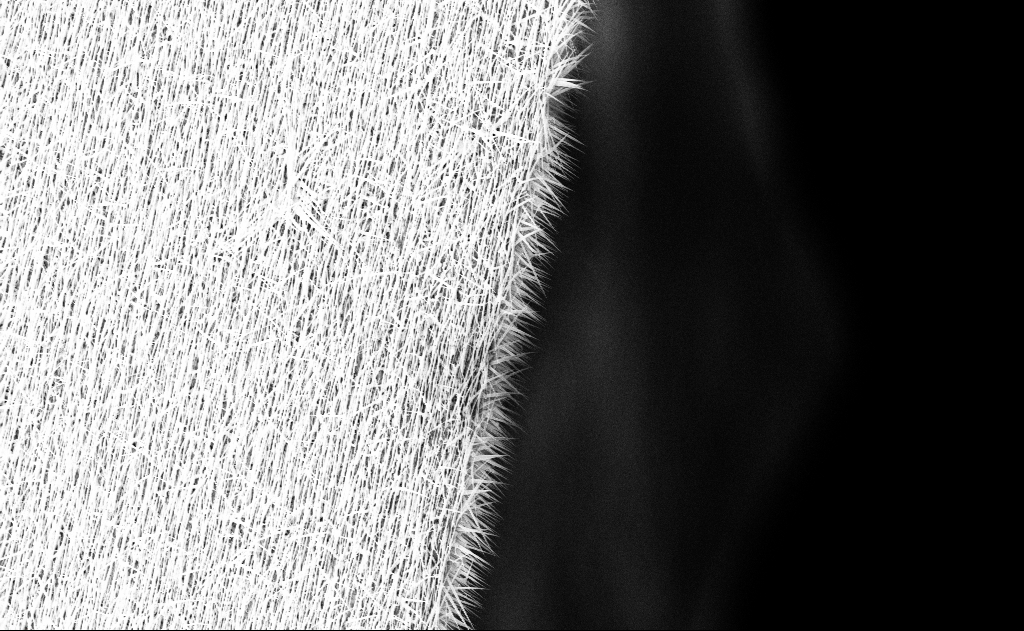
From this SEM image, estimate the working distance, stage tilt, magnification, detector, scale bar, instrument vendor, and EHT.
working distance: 16 mm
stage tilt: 0°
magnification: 5 K X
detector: InLens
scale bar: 10000 nm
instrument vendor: Zeiss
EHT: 10 kV